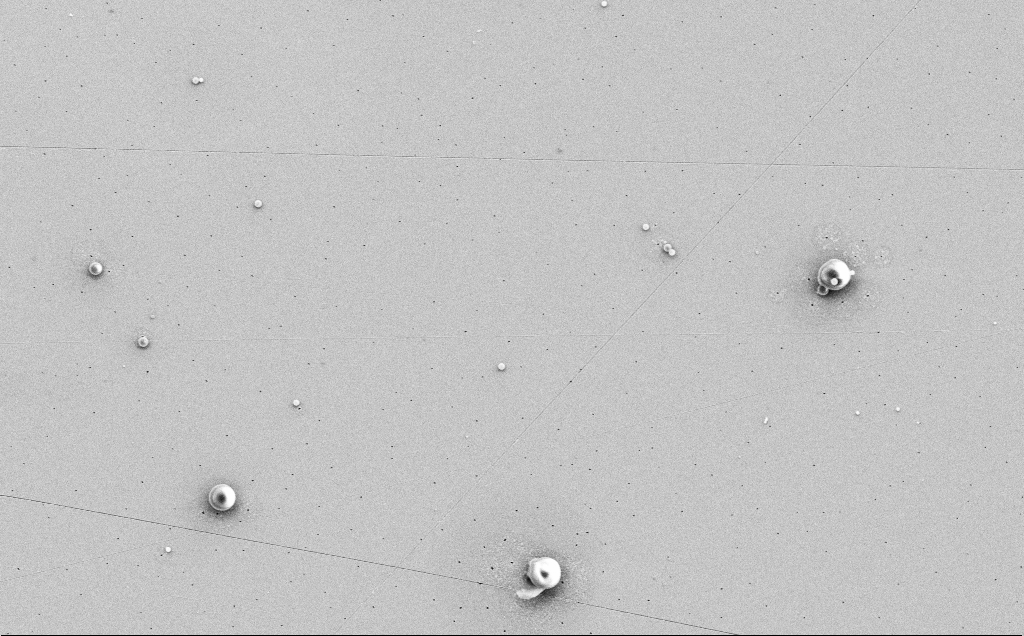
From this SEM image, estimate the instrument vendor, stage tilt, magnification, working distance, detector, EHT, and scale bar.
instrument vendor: Zeiss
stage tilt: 0°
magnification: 4.2 K X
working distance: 12 mm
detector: SE2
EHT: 5 kV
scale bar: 10000 nm